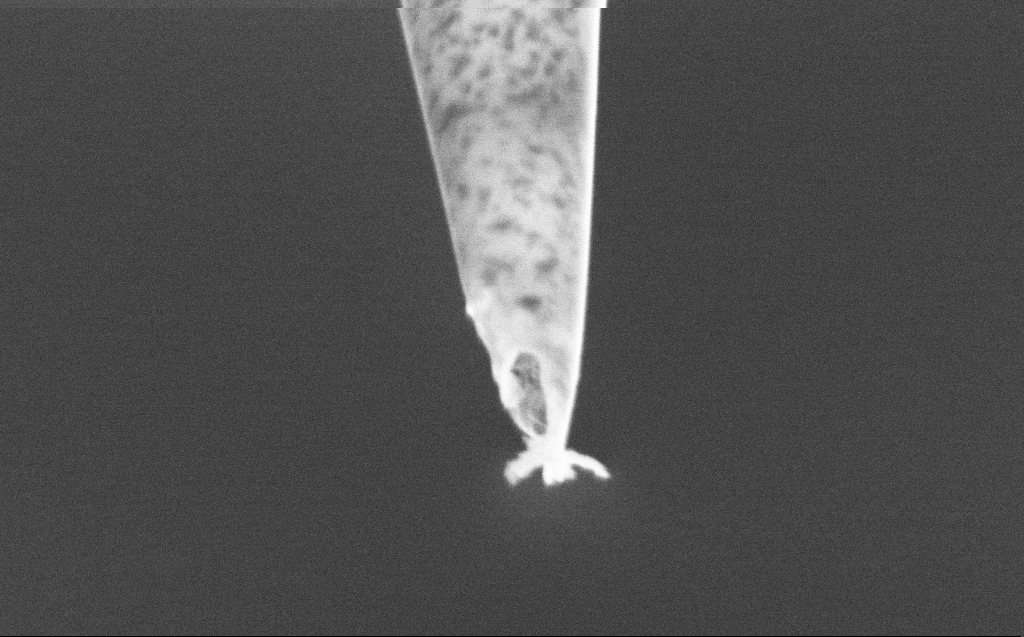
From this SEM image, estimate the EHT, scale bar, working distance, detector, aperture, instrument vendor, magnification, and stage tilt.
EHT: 2 kV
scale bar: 200 nm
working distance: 6 mm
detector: SE2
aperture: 30 µm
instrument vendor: Zeiss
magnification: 100 K X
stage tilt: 45°